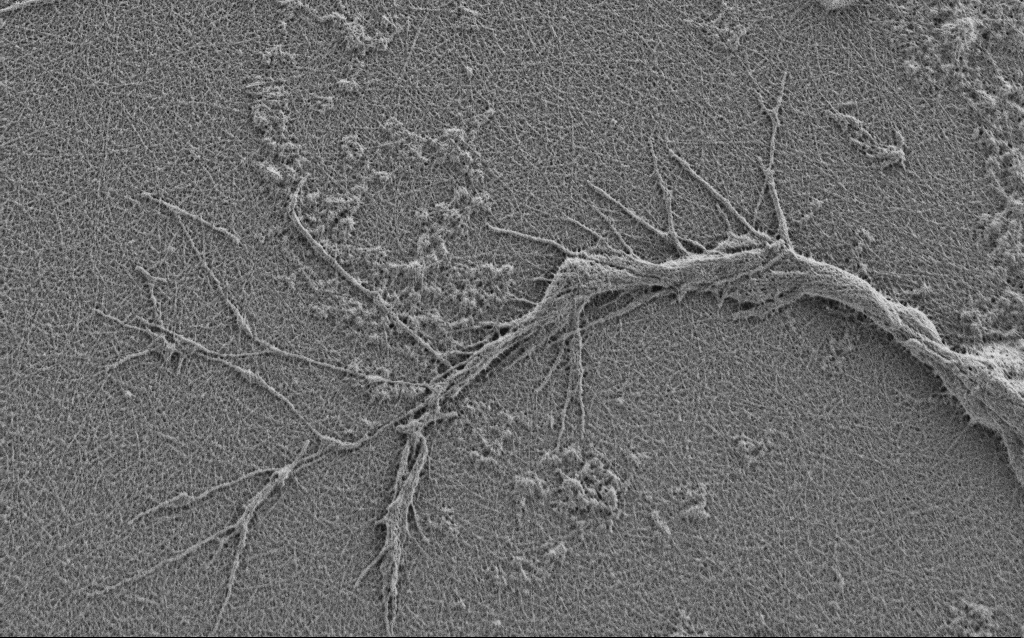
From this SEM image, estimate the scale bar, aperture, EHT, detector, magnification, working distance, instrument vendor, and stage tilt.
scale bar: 2000 nm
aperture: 30 µm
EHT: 0.9 kV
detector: SE2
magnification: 10 K X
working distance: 4 mm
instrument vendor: Zeiss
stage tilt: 0°